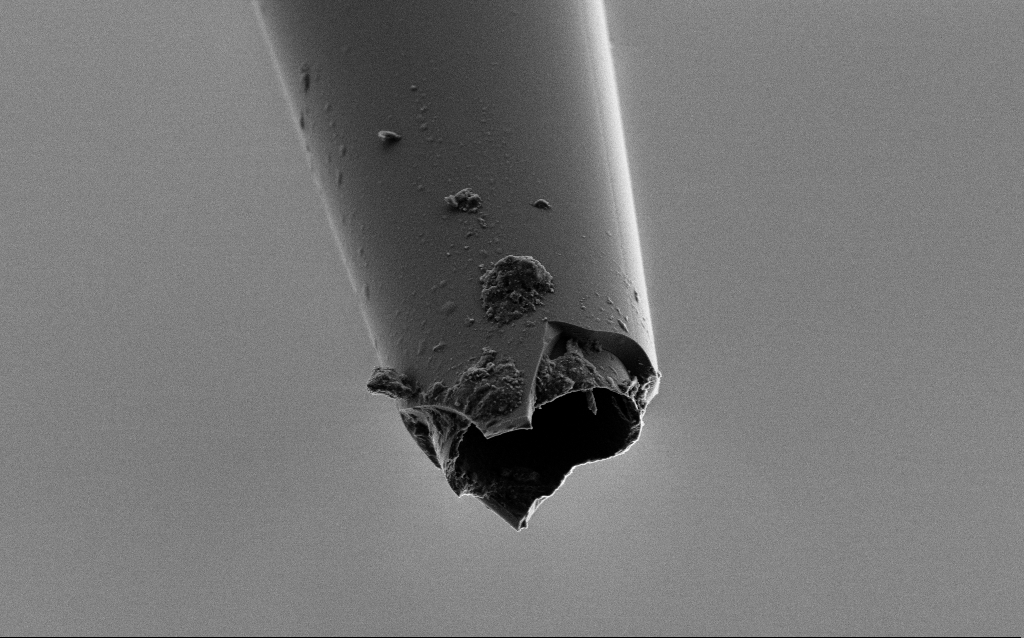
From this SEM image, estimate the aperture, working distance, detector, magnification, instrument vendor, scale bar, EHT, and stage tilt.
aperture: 30 µm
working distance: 6 mm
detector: SE2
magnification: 10 K X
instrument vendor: Zeiss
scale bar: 2000 nm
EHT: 2 kV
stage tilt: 45°